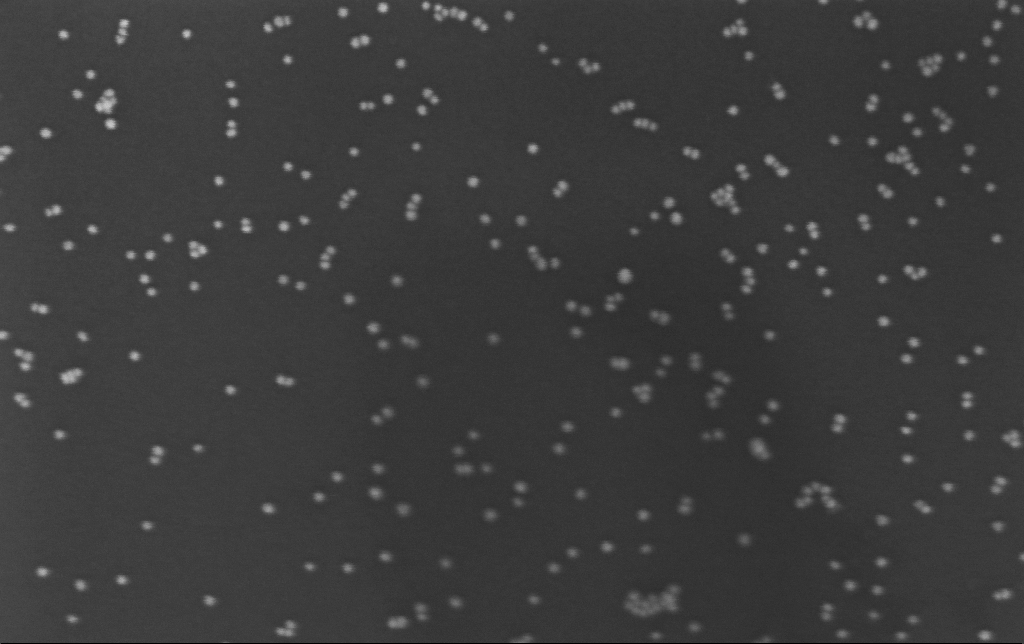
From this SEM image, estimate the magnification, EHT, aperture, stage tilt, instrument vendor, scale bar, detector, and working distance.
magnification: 234.43 K X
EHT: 3 kV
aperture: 30 µm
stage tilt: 0°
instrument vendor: Zeiss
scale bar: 200 nm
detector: InLens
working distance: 3.2 mm